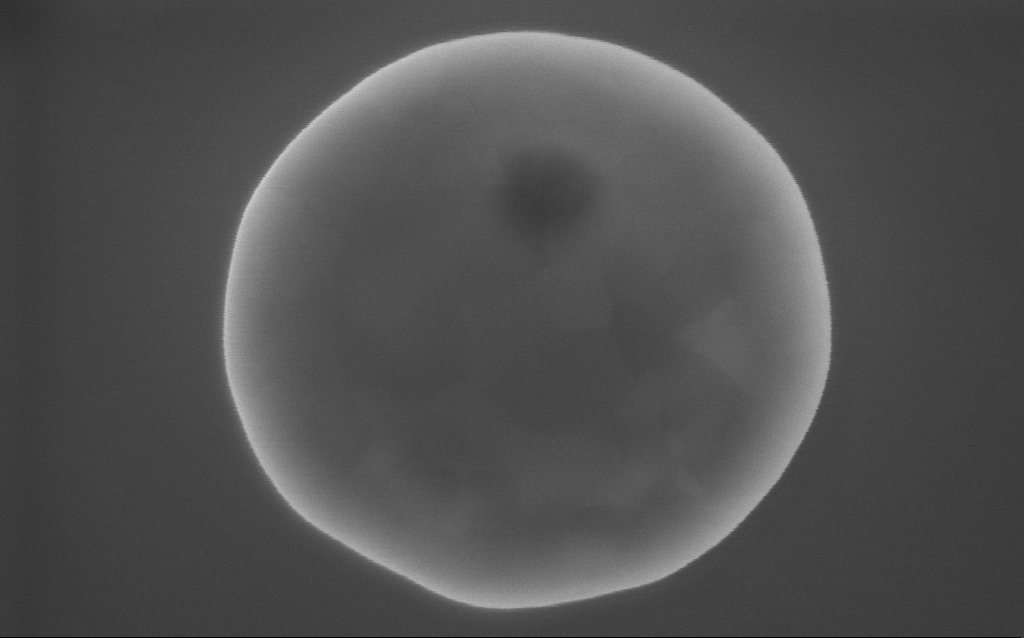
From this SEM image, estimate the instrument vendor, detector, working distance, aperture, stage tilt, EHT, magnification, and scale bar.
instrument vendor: Zeiss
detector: InLens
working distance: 2 mm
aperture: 30 µm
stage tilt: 0°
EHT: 10 kV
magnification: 175 K X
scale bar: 200 nm